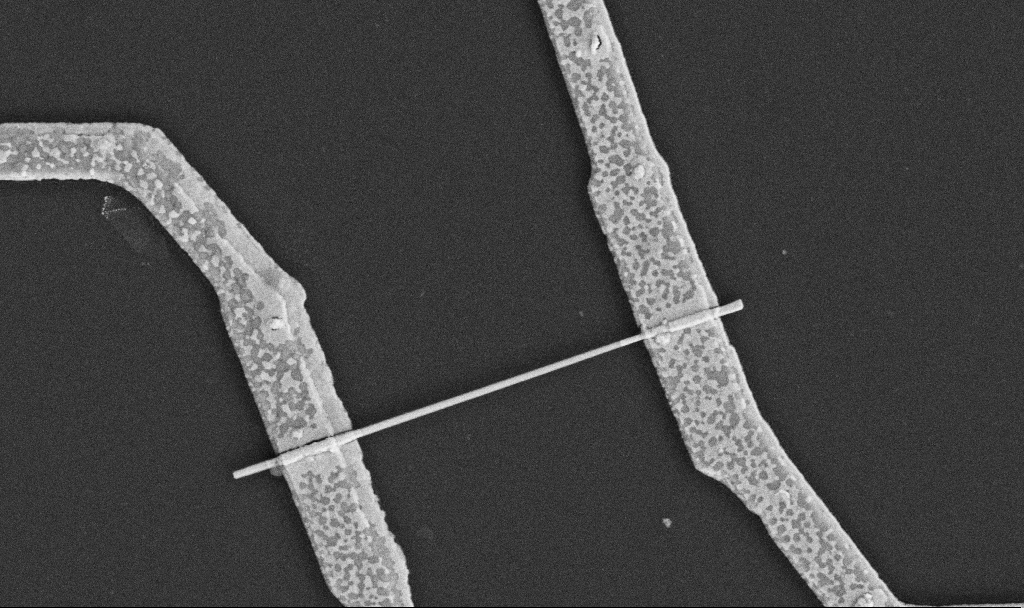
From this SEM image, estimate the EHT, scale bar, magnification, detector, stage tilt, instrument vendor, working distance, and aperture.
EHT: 5 kV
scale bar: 1000 nm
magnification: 30 K X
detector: SE2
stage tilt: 0°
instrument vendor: Zeiss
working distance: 10.6 mm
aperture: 30 µm